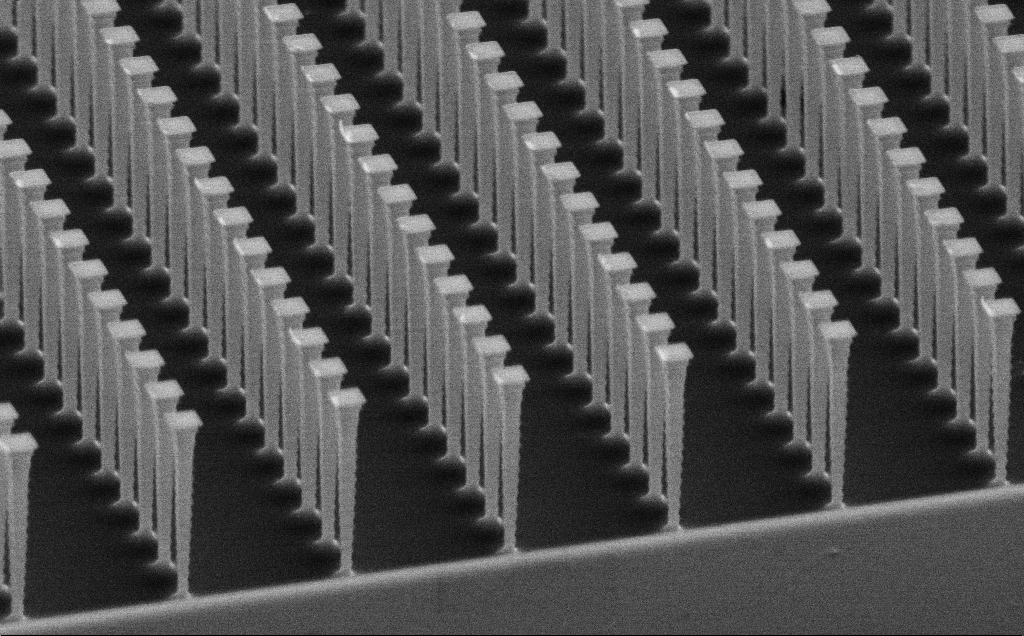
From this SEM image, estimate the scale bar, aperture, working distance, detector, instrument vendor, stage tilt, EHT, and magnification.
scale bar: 1000 nm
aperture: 30 µm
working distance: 8 mm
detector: SE2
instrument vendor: Zeiss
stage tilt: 62°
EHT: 10 kV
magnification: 12.58 K X